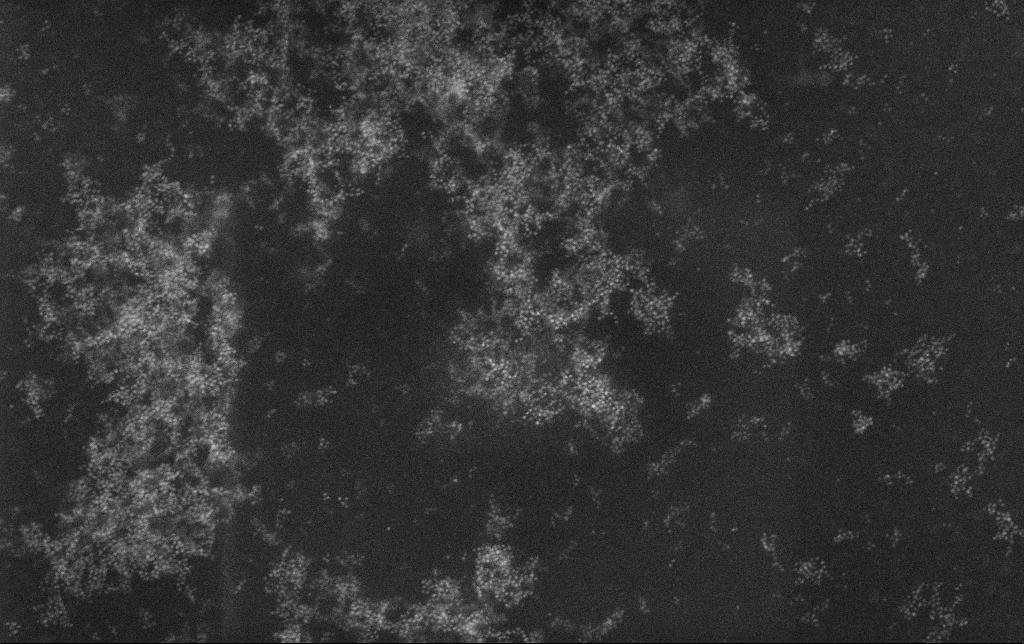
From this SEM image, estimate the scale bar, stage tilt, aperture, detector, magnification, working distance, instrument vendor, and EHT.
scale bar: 200 nm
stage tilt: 0°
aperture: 30 µm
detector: InLens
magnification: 100 K X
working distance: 3.4 mm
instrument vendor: Zeiss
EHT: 10 kV